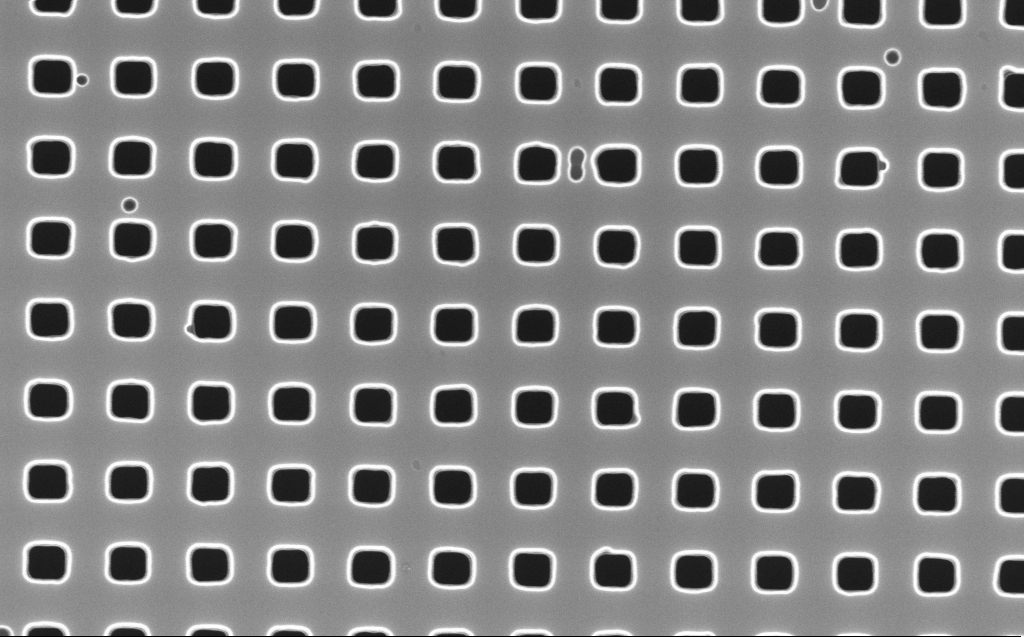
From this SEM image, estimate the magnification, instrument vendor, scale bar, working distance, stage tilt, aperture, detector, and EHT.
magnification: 60 K X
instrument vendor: Zeiss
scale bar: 1000 nm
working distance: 6 mm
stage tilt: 0°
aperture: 30 µm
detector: InLens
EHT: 10 kV